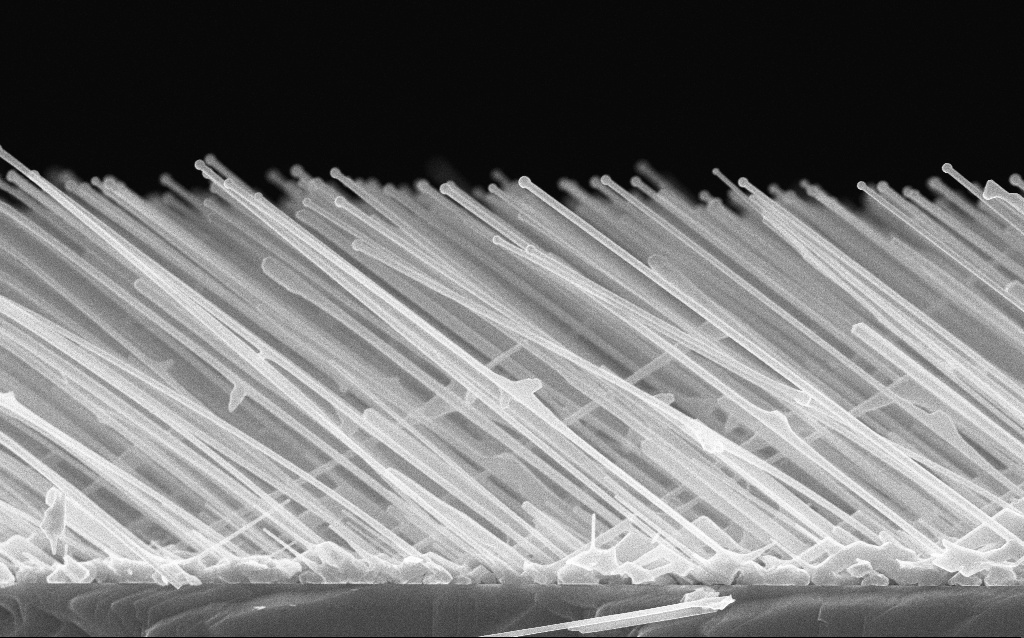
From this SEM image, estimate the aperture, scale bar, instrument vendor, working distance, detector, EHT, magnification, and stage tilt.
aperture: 30 µm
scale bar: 1000 nm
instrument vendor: Zeiss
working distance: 4 mm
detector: InLens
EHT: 10 kV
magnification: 25 K X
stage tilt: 0°